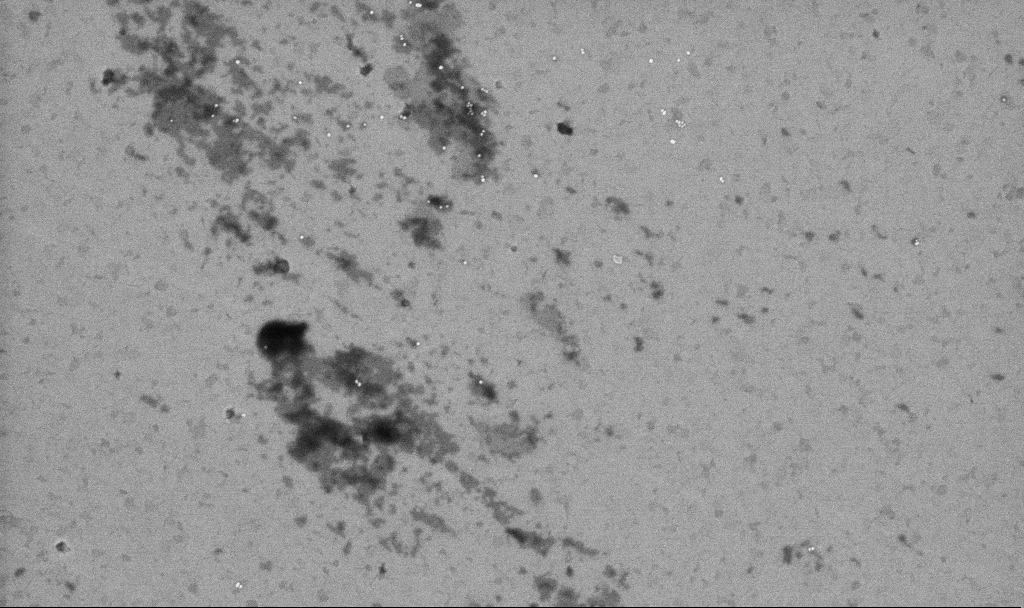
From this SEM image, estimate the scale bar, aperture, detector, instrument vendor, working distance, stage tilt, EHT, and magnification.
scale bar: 1000 nm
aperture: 30 µm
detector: InLens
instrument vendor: Zeiss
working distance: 3.3 mm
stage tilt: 0°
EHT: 10 kV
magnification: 50.38 K X